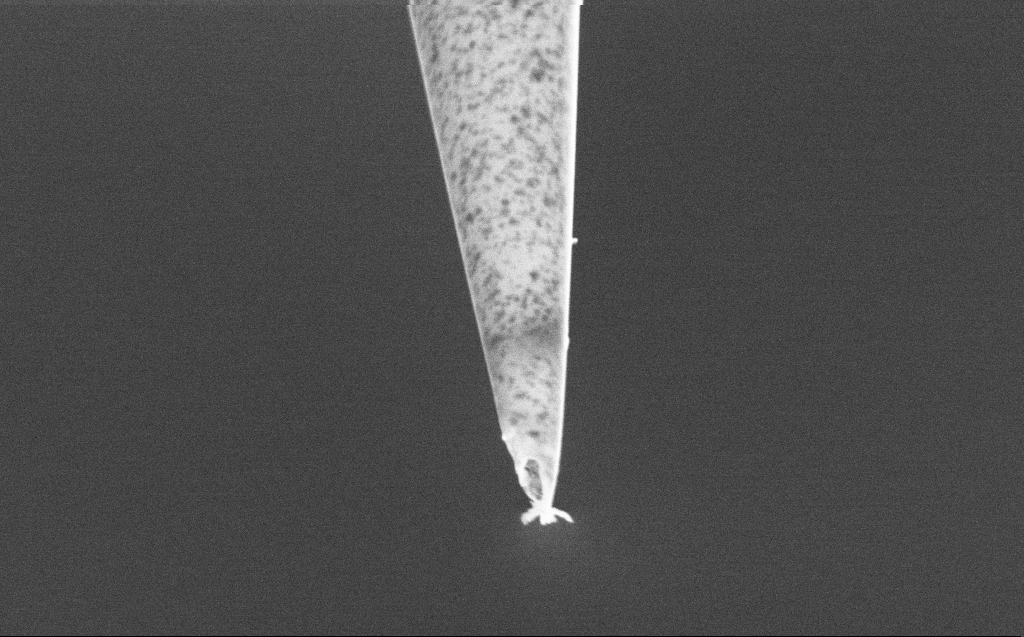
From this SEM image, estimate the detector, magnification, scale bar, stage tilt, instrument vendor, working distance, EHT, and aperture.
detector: SE2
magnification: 50 K X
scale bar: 1000 nm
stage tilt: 45°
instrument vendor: Zeiss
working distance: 6 mm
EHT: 2 kV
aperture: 30 µm